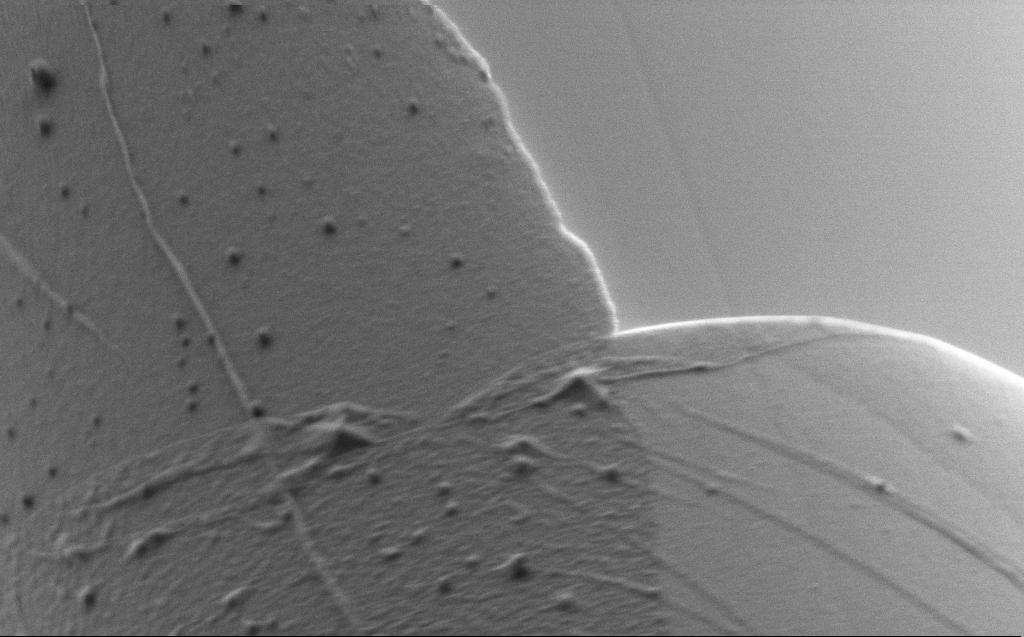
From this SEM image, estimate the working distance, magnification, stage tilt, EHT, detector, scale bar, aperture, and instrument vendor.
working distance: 4 mm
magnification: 25 K X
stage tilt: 45°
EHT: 1 kV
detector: SE2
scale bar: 2000 nm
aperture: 30 µm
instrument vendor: Zeiss